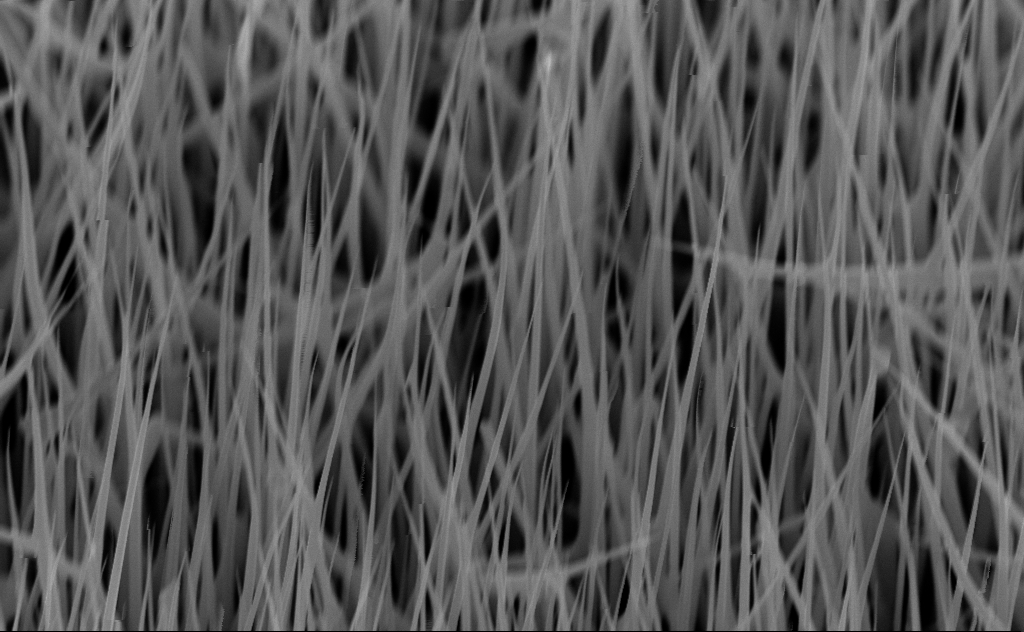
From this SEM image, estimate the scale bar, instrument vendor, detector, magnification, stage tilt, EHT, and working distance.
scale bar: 1000 nm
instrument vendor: Zeiss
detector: InLens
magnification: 40 K X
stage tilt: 45°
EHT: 10 kV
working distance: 7 mm